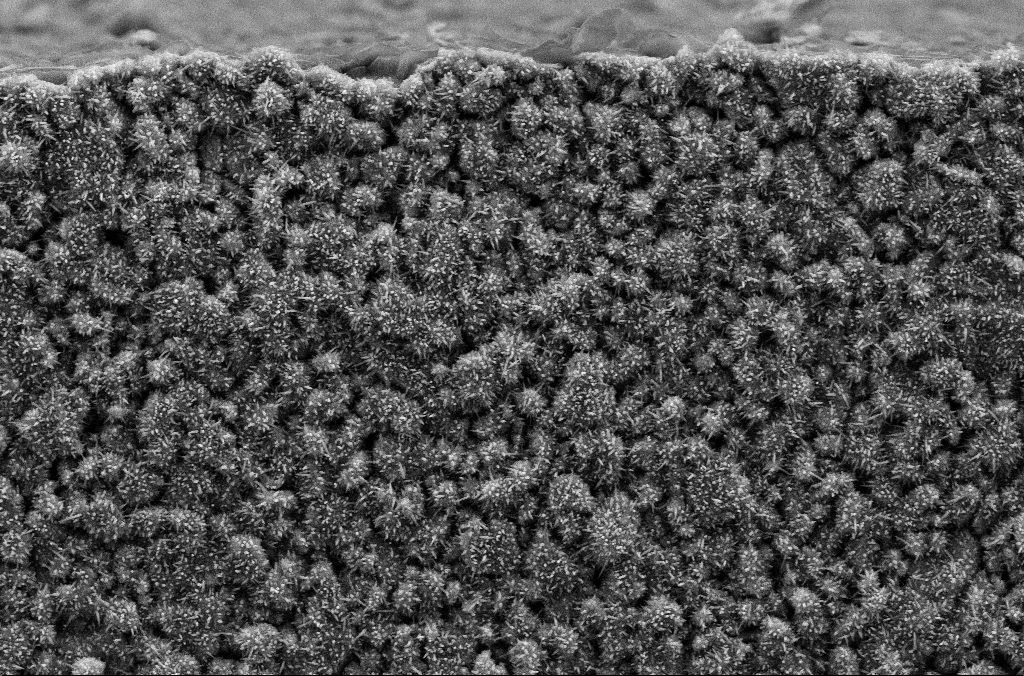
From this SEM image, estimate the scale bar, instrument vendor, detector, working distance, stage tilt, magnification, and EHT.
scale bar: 1000 nm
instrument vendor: Zeiss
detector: SE2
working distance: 5.2 mm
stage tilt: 0.1°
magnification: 15.15 K X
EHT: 10 kV